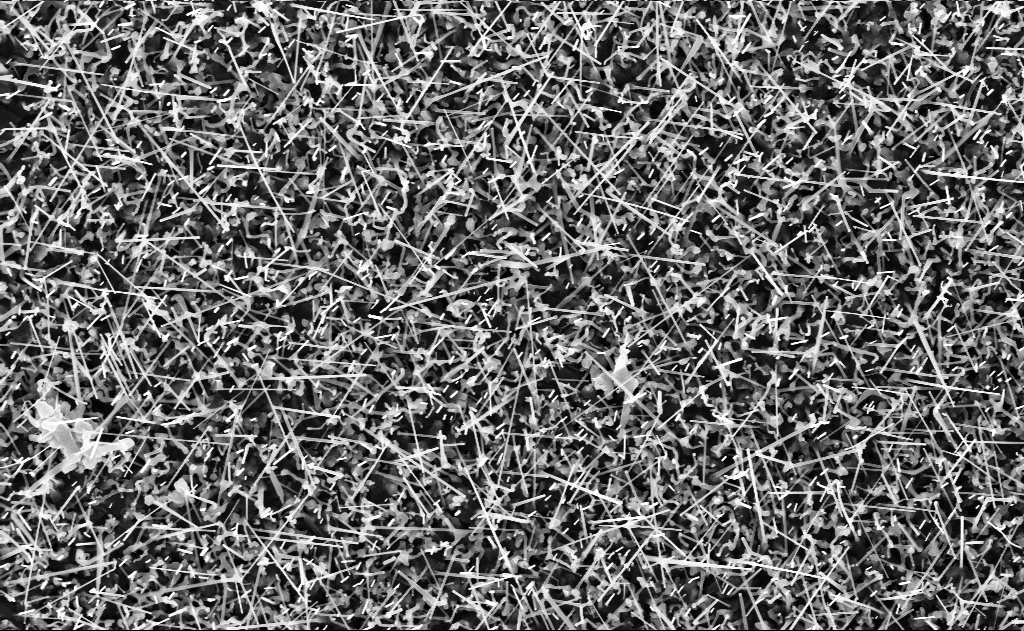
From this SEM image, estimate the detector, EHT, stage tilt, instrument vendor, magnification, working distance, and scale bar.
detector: InLens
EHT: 10 kV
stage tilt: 0°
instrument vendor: Zeiss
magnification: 20 K X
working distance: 10 mm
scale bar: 1000 nm